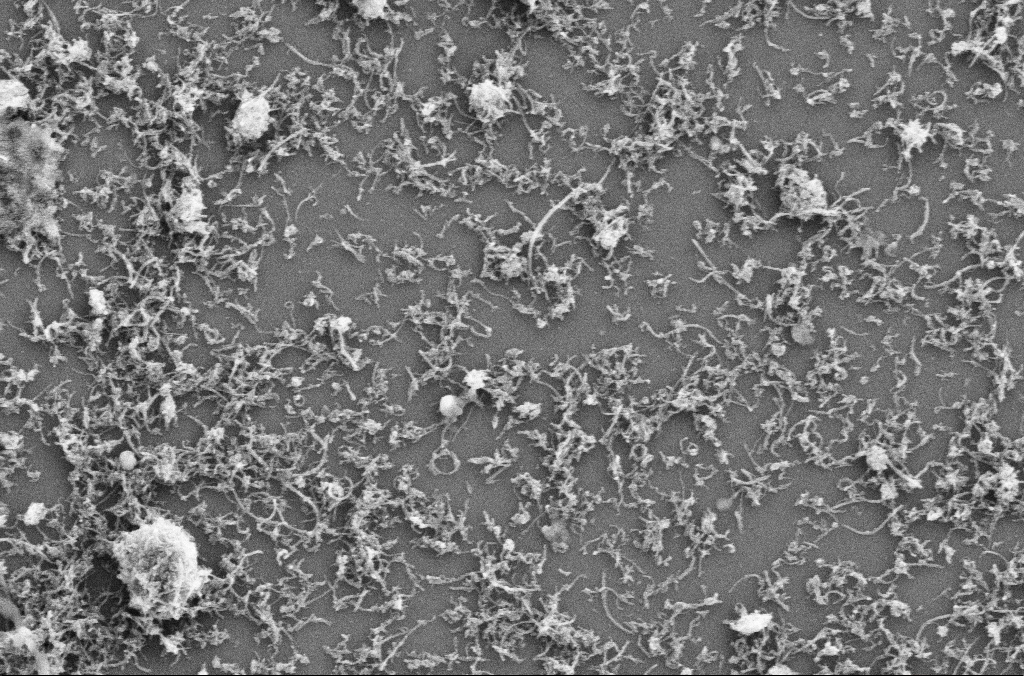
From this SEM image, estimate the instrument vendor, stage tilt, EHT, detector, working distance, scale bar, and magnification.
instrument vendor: Zeiss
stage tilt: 0°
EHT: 5 kV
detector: SE2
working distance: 4 mm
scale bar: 1000 nm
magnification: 20 K X